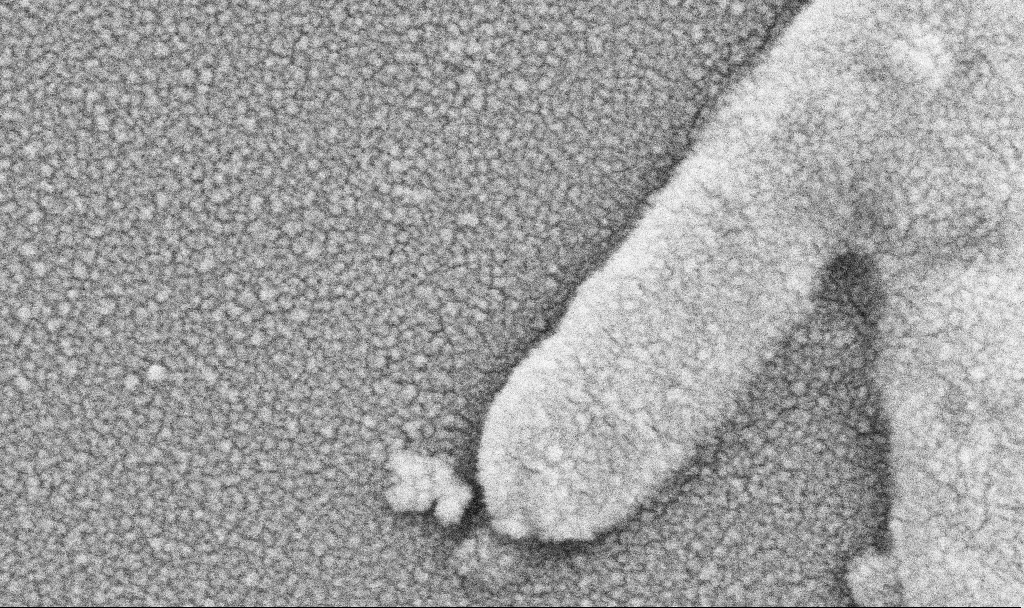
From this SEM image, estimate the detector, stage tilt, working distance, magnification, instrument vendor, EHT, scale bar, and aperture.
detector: SE2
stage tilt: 0°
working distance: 10.7 mm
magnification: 162.23 K X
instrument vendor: Zeiss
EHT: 5 kV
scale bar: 200 nm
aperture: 30 µm